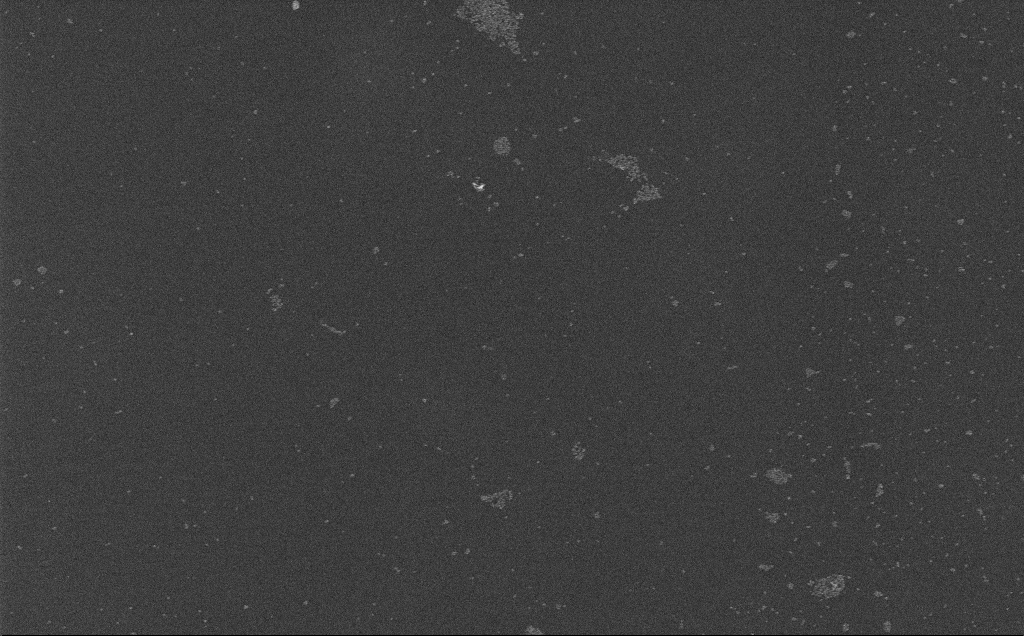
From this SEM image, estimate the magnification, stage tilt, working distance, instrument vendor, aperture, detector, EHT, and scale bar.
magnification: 27.55 K X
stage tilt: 0°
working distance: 3.7 mm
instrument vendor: Zeiss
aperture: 30 µm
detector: InLens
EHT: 10 kV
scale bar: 2000 nm